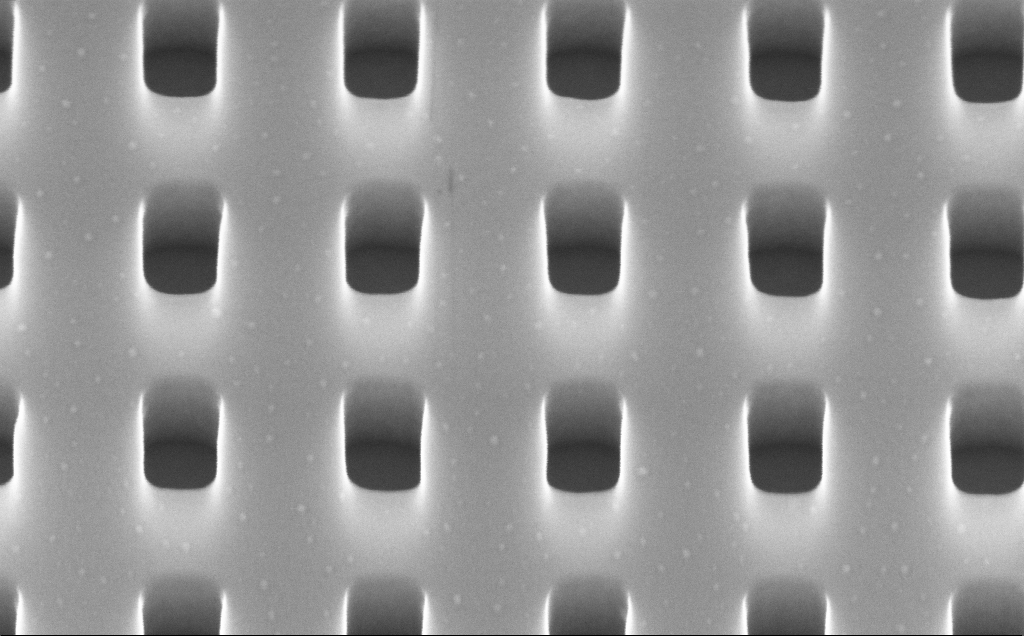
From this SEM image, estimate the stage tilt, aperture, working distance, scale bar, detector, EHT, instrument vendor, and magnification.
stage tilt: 45°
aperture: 30 µm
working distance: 5 mm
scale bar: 200 nm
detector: InLens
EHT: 10 kV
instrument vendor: Zeiss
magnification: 150 K X